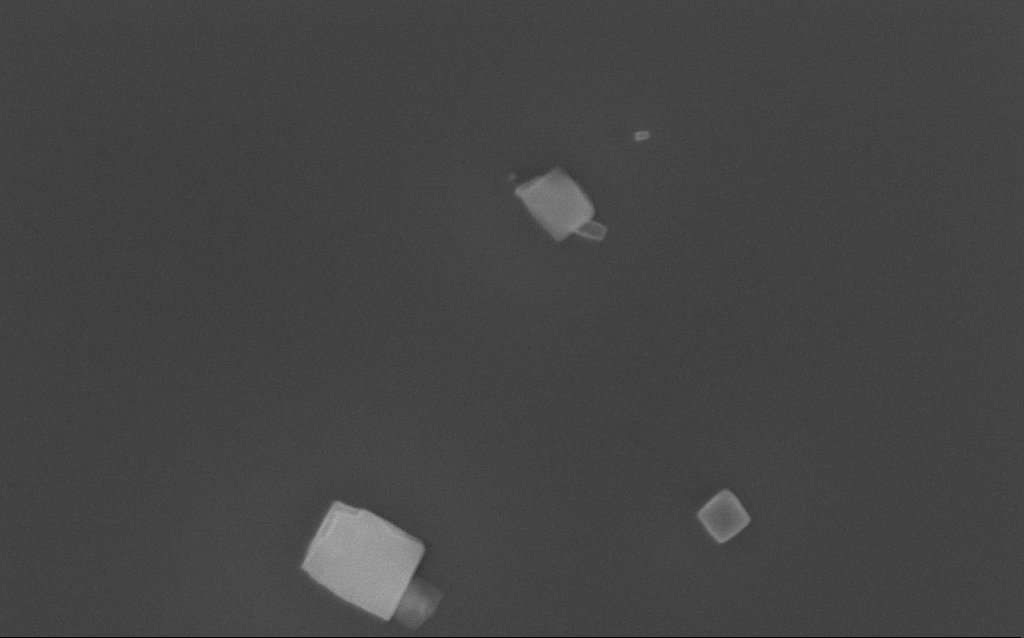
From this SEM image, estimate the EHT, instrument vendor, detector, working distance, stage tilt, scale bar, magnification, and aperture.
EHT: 10 kV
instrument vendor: Zeiss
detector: InLens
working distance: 3 mm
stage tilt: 0°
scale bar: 200 nm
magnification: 79.34 K X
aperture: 30 µm